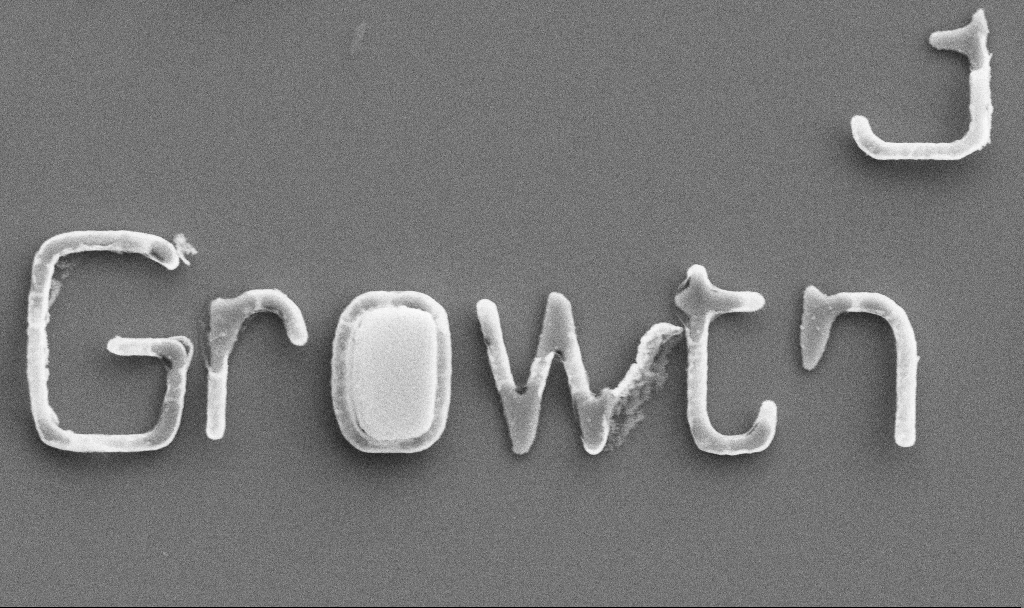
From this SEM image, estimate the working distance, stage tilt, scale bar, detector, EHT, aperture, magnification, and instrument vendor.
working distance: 10.7 mm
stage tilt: -0°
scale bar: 1000 nm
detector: SE2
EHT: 5 kV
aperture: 30 µm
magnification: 20 K X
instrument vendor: Zeiss